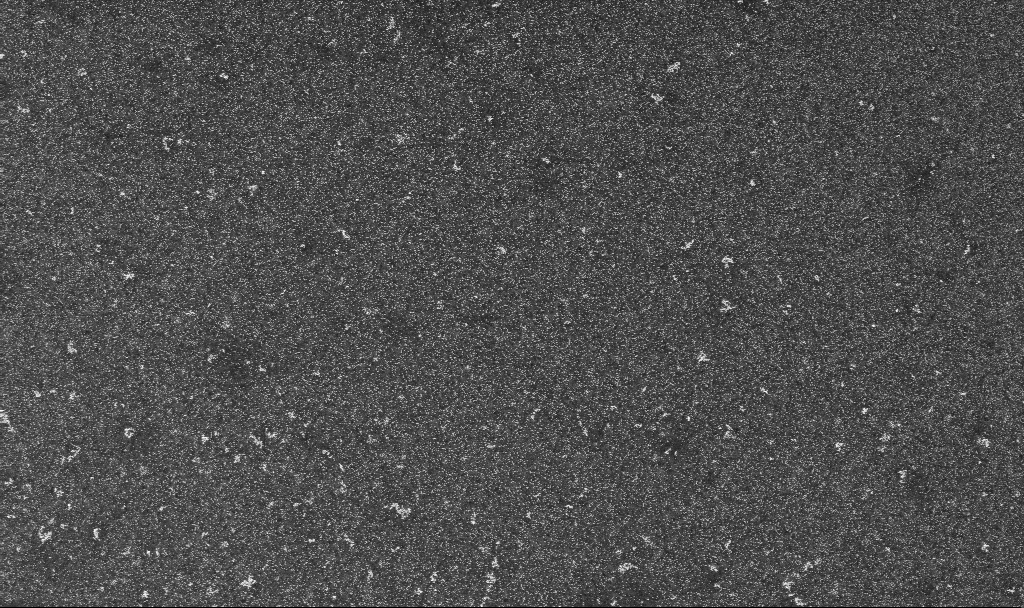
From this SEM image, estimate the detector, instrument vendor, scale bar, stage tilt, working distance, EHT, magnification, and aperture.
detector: InLens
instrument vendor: Zeiss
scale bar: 10000 nm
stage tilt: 0°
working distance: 3.3 mm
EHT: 10 kV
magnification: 5 K X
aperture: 30 µm